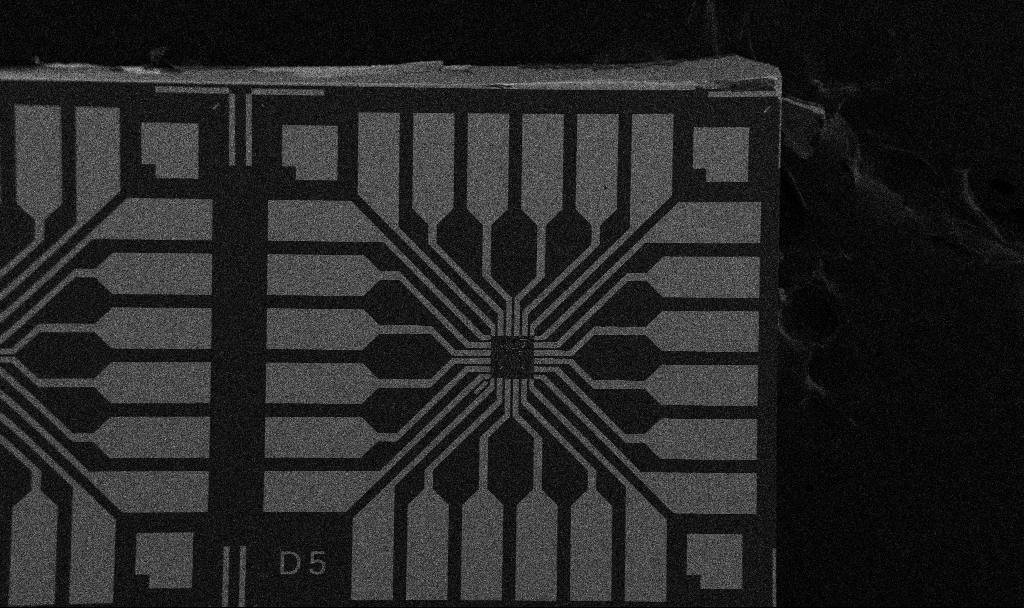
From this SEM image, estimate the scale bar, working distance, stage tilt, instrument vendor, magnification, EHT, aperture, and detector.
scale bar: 200000 nm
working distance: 10.7 mm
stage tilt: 0°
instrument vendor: Zeiss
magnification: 0.1 K X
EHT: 5 kV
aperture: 30 µm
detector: SE2